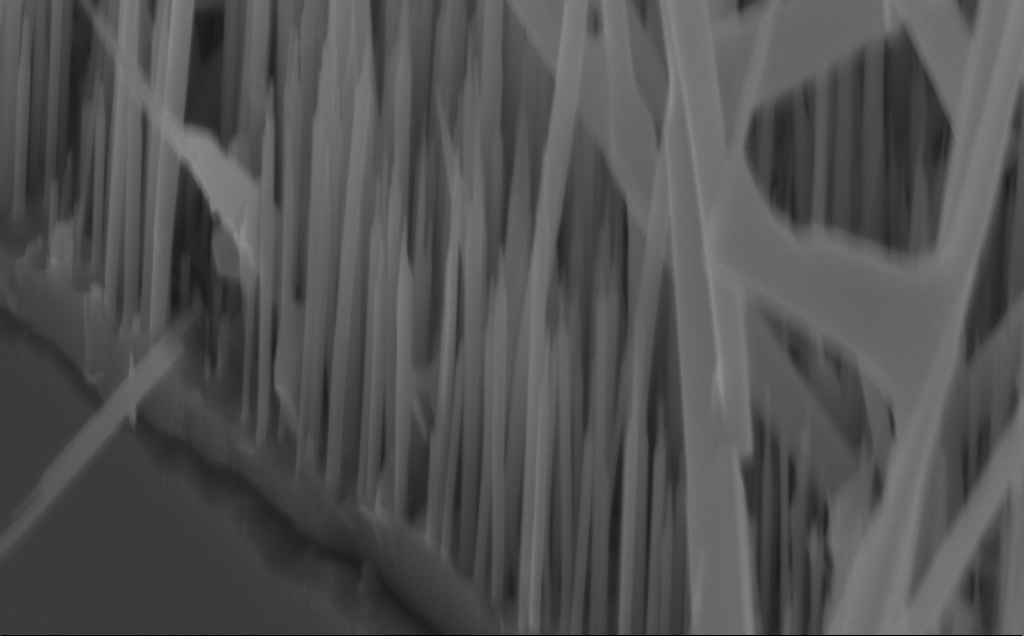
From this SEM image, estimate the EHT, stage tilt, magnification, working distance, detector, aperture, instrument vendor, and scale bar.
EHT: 10 kV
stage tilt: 45°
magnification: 75.95 K X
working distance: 5 mm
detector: InLens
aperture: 30 µm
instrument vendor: Zeiss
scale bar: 200 nm